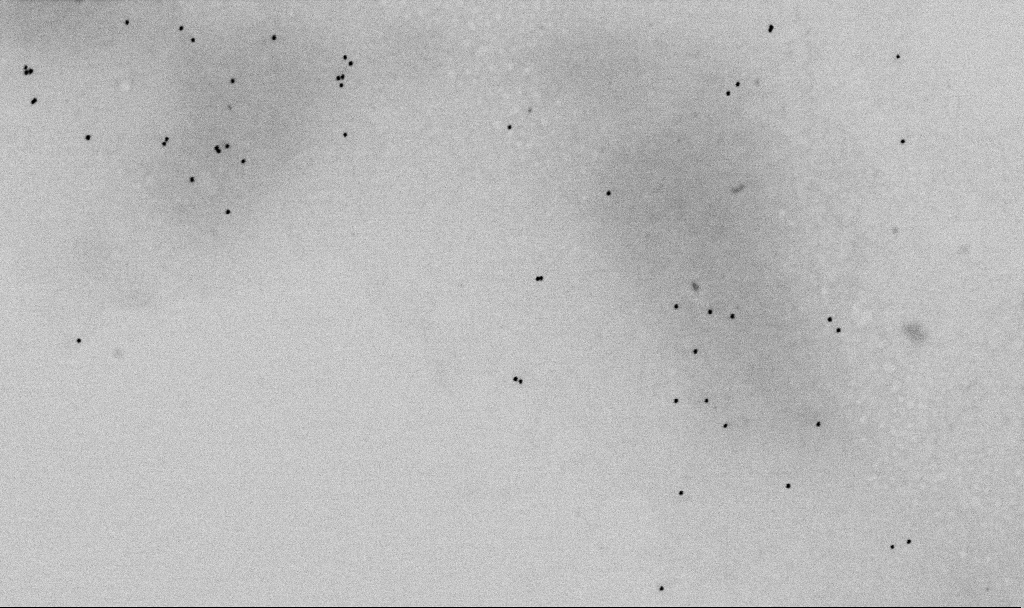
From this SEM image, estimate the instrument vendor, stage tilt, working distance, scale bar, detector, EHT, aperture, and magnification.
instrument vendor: Zeiss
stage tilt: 0°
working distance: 5.5 mm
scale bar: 1000 nm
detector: SE2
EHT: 2 kV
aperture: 30 µm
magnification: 60 K X